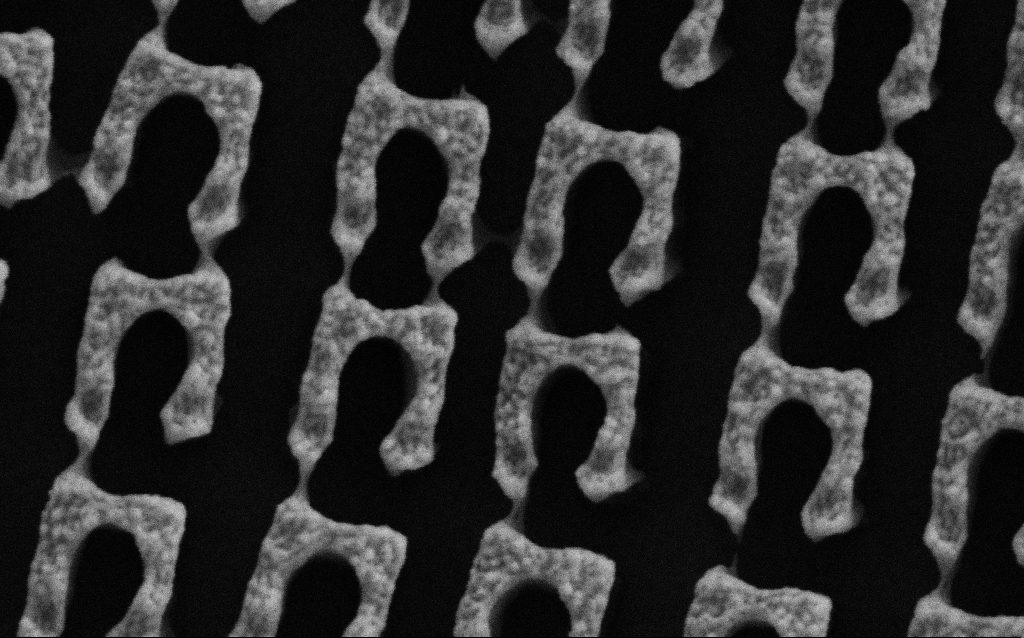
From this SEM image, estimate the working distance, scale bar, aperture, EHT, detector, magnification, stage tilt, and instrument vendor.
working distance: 4.6 mm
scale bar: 200 nm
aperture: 30 µm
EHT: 3 kV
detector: SE2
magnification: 175.6 K X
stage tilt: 0°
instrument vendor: Zeiss